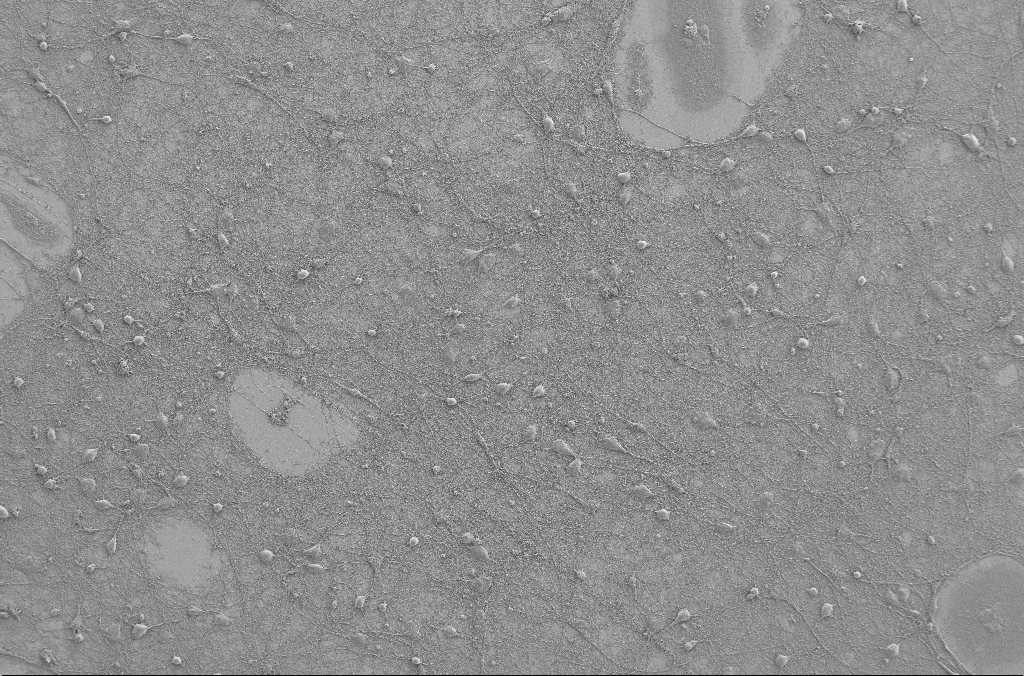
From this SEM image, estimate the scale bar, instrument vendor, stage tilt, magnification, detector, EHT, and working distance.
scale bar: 100000 nm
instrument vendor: Zeiss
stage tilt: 0°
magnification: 0.5 K X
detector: SE2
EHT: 2 kV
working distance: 4 mm